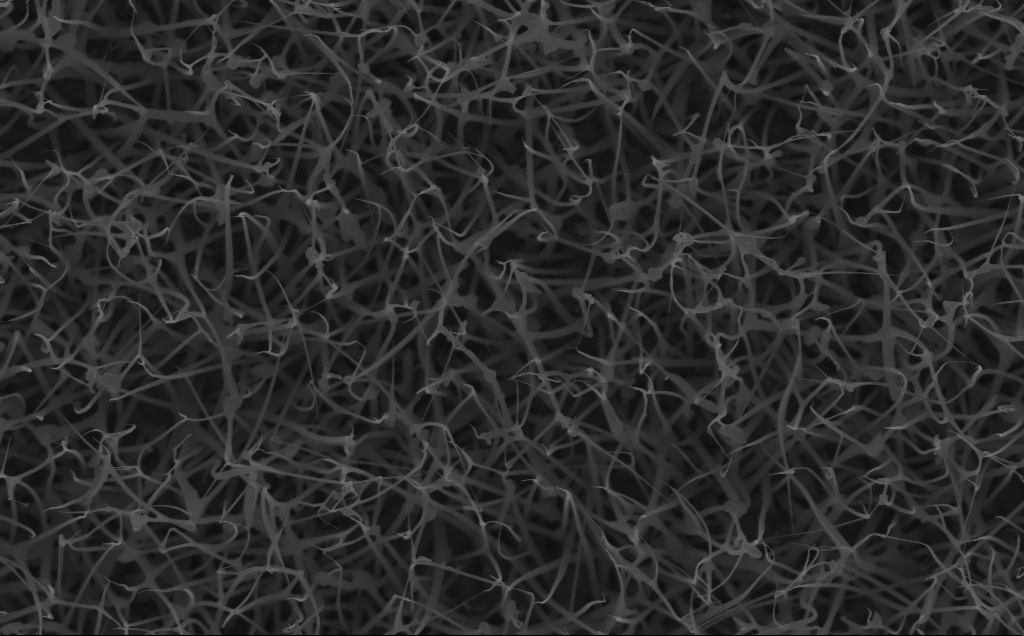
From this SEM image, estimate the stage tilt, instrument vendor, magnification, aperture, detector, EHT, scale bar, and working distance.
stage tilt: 0°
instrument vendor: Zeiss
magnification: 20 K X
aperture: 30 µm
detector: InLens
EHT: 10 kV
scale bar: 2000 nm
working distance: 6 mm